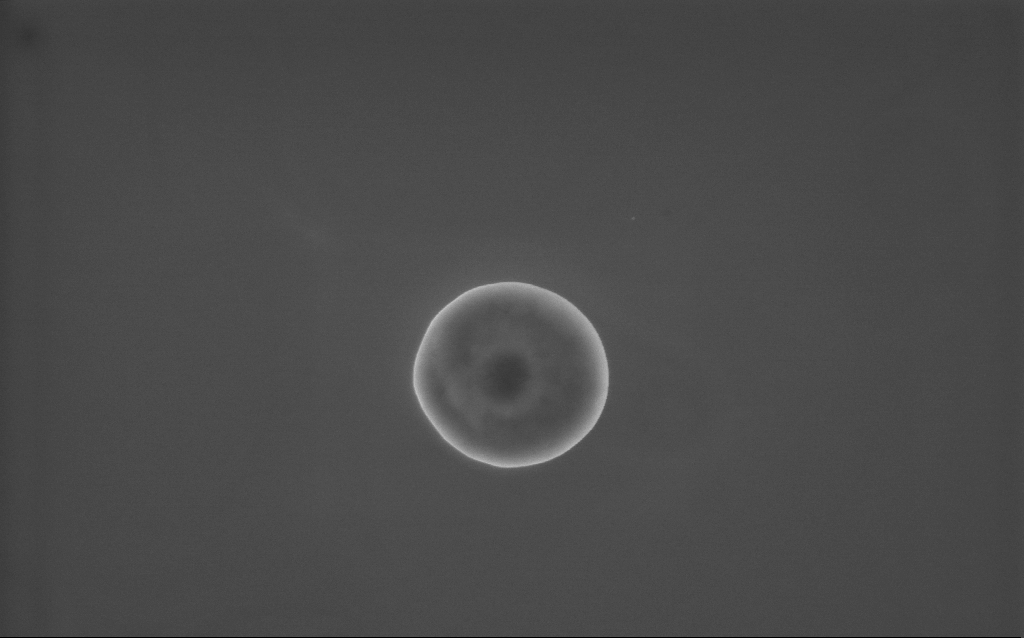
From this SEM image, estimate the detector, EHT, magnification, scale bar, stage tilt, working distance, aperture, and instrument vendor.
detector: InLens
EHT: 10 kV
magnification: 57 K X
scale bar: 1000 nm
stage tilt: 0°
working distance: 3 mm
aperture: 30 µm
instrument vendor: Zeiss